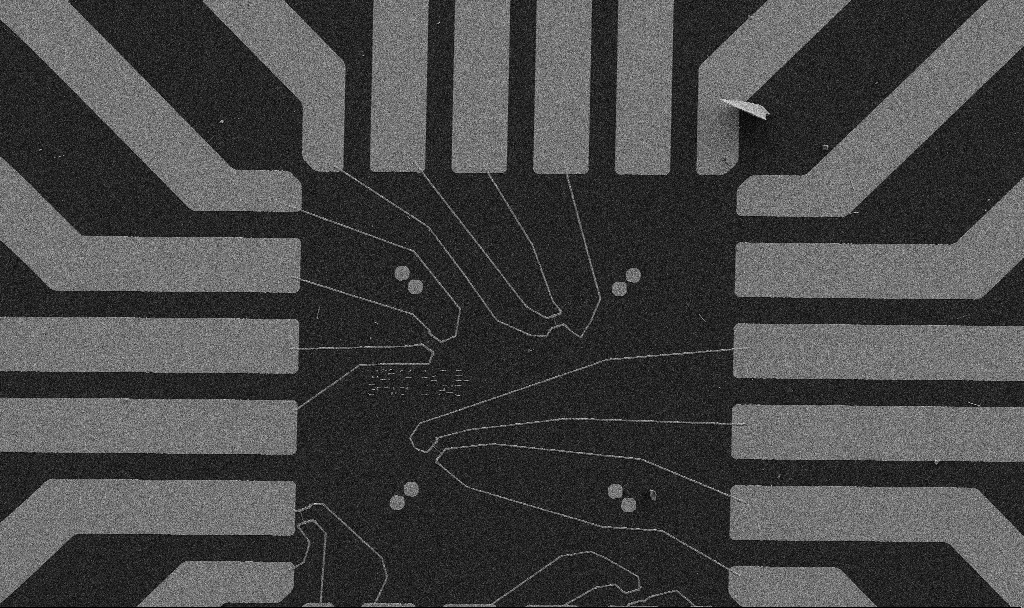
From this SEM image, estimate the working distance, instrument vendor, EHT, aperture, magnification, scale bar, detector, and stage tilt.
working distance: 6.7 mm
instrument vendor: Zeiss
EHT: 5 kV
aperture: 30 µm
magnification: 1 K X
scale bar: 20000 nm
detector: SE2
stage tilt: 0°